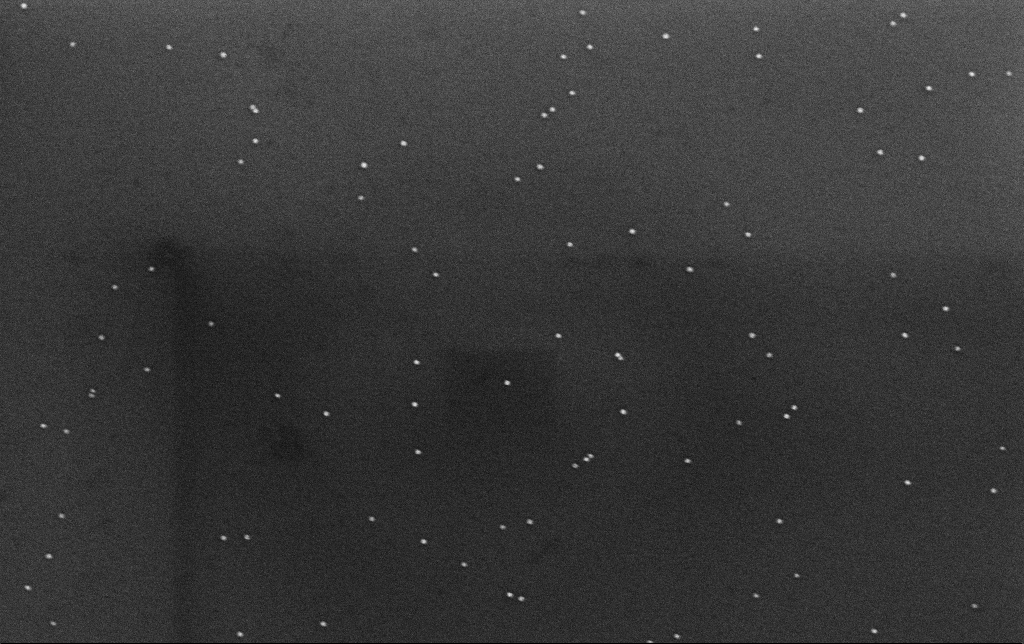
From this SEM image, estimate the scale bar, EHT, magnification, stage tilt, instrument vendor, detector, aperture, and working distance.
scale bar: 200 nm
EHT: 10 kV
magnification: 100 K X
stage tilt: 0°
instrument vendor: Zeiss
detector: InLens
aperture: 30 µm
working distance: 3.1 mm